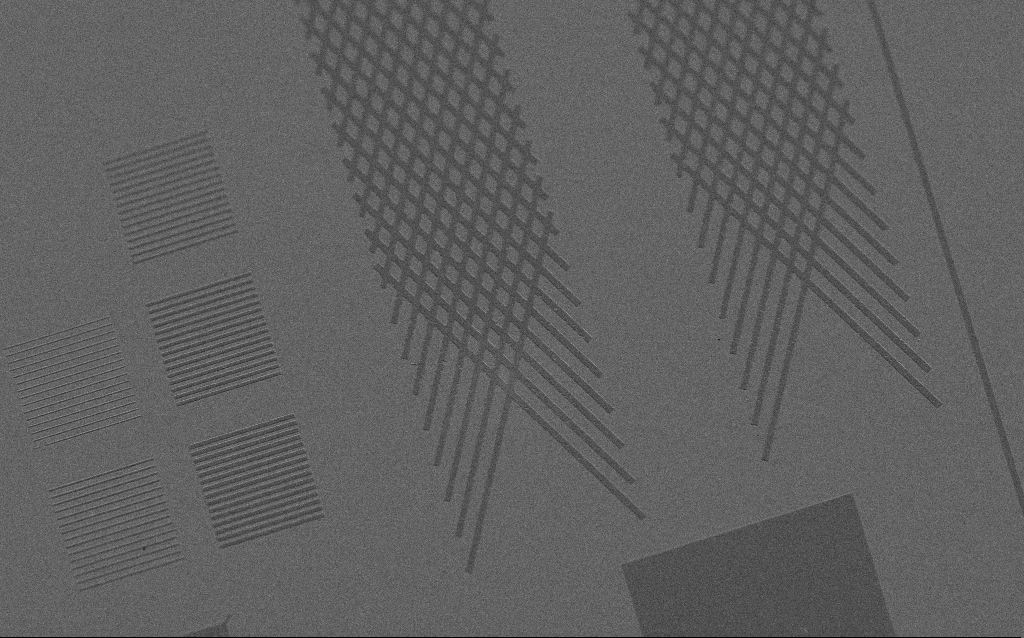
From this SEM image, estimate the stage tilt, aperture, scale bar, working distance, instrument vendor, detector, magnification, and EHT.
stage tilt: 45°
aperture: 30 µm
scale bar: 10000 nm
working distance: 4 mm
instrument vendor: Zeiss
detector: SE2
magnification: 2.74 K X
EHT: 2 kV